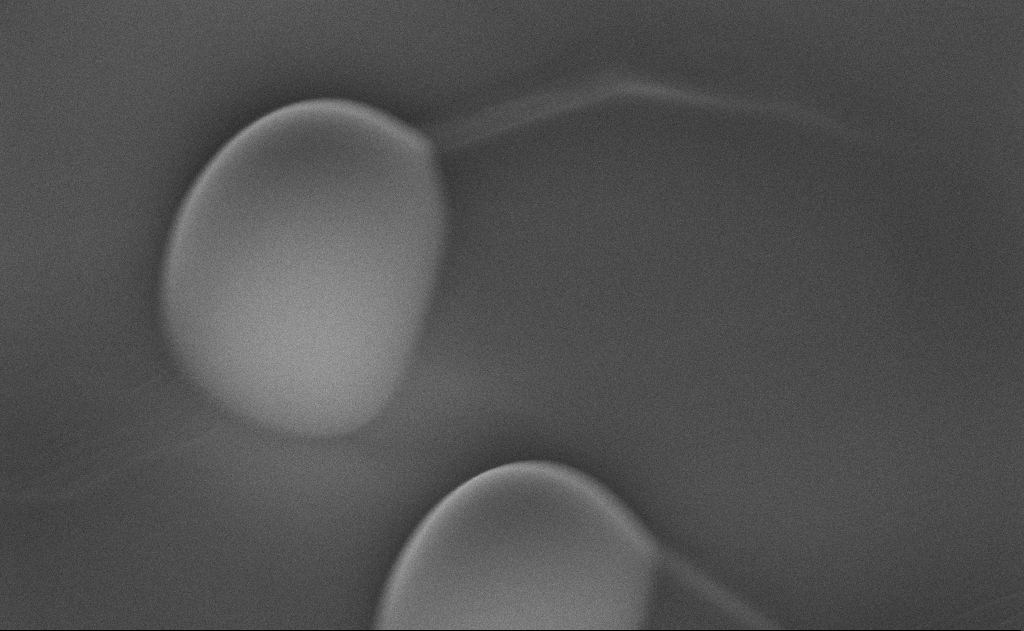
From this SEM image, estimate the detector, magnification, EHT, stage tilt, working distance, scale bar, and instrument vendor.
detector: InLens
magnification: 95.69 K X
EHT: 10 kV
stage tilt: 48.9°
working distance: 7 mm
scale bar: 200 nm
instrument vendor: Zeiss